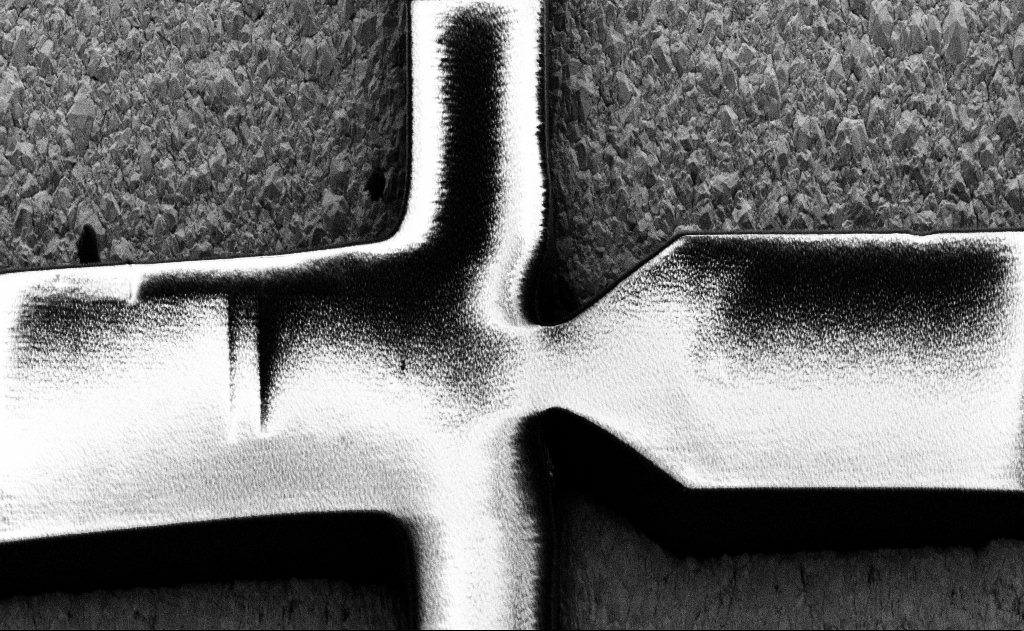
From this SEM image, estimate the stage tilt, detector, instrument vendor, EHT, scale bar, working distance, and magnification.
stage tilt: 25°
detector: SE2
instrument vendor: Zeiss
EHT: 3 kV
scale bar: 10000 nm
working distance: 10 mm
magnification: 5.51 K X